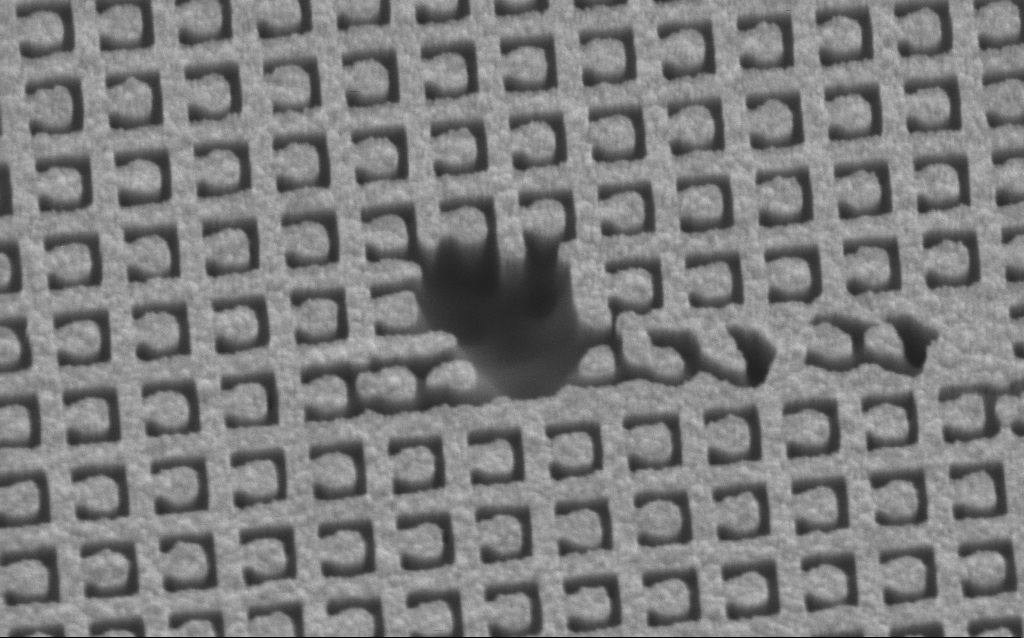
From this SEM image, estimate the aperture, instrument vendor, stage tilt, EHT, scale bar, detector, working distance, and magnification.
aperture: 30 µm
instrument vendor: Zeiss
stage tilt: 45°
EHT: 3 kV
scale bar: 1000 nm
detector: SE2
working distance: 7.8 mm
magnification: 63.29 K X